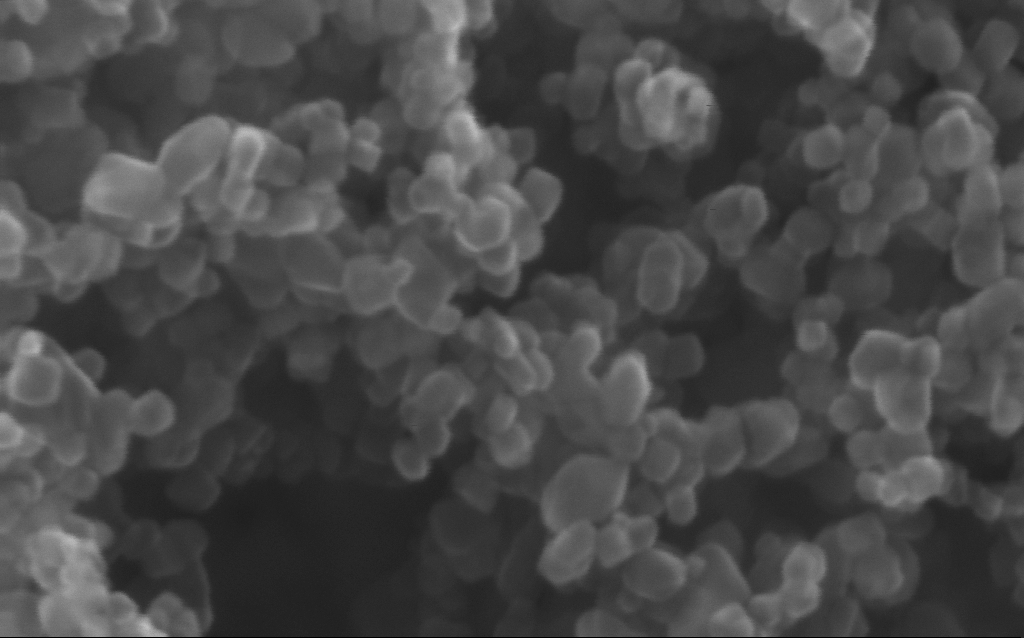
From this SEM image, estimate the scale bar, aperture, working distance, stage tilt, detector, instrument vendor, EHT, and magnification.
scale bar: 100 nm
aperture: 30 µm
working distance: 3 mm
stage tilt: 0°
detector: InLens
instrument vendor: Zeiss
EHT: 20 kV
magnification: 716 K X